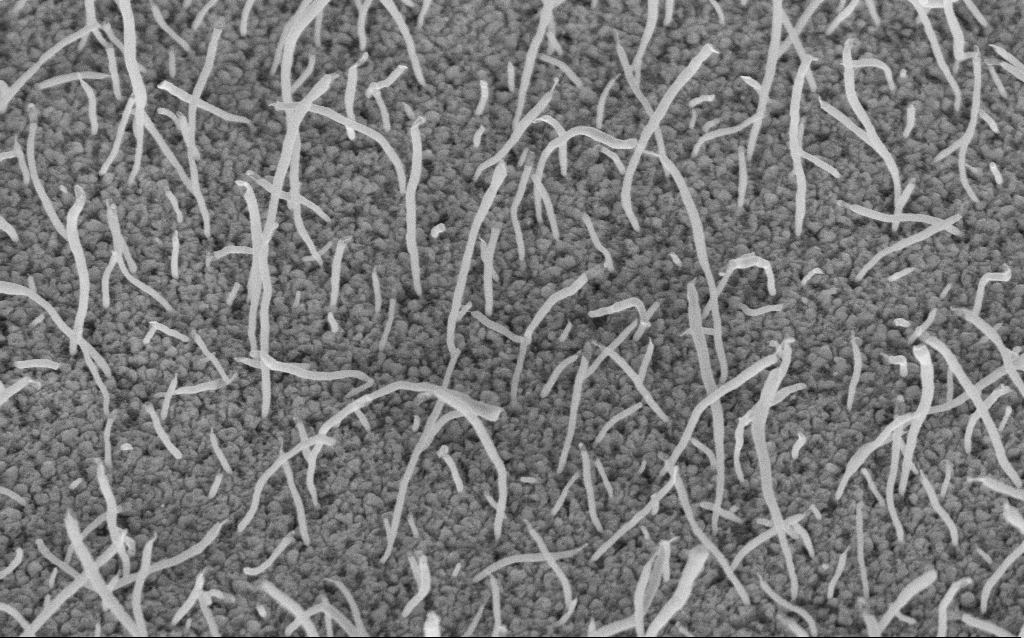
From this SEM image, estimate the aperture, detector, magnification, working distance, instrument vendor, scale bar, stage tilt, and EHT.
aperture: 30 µm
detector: InLens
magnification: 50 K X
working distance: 8.7 mm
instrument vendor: Zeiss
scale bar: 1000 nm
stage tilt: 45°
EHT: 5 kV